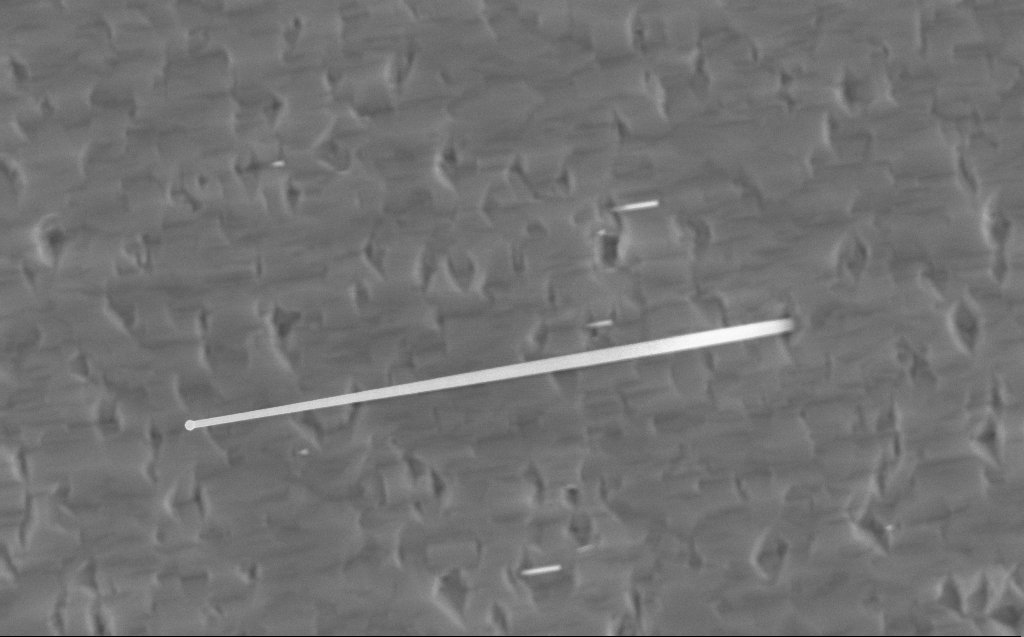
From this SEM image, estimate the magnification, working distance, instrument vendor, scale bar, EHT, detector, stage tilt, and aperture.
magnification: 40 K X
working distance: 3 mm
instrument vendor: Zeiss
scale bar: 1000 nm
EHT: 10 kV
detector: InLens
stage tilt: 0°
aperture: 30 µm